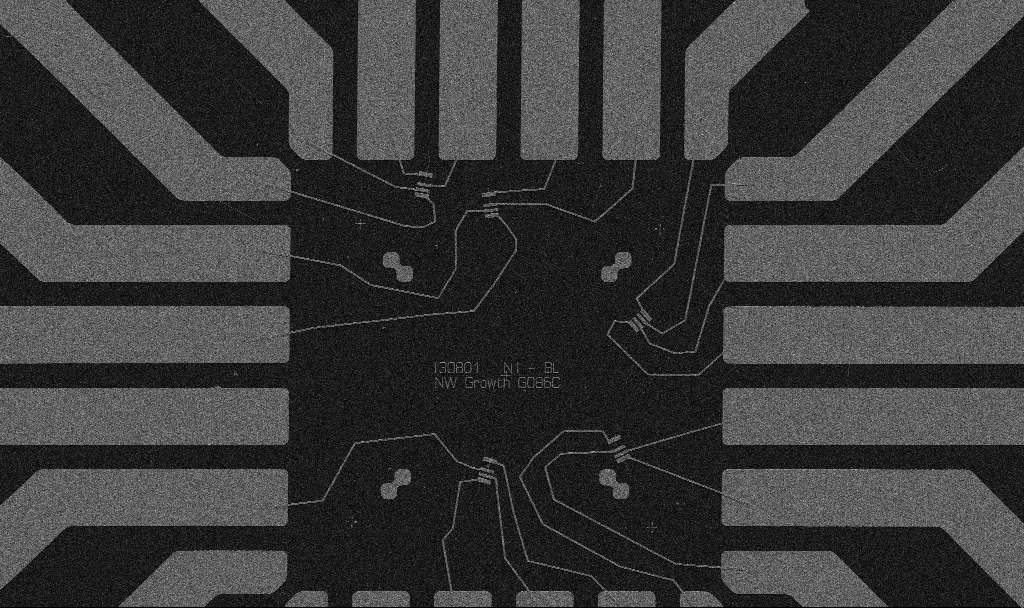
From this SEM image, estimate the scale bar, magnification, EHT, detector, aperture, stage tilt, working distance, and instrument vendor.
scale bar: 20000 nm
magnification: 1 K X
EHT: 5 kV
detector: SE2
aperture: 30 µm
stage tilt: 0°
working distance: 10.7 mm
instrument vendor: Zeiss